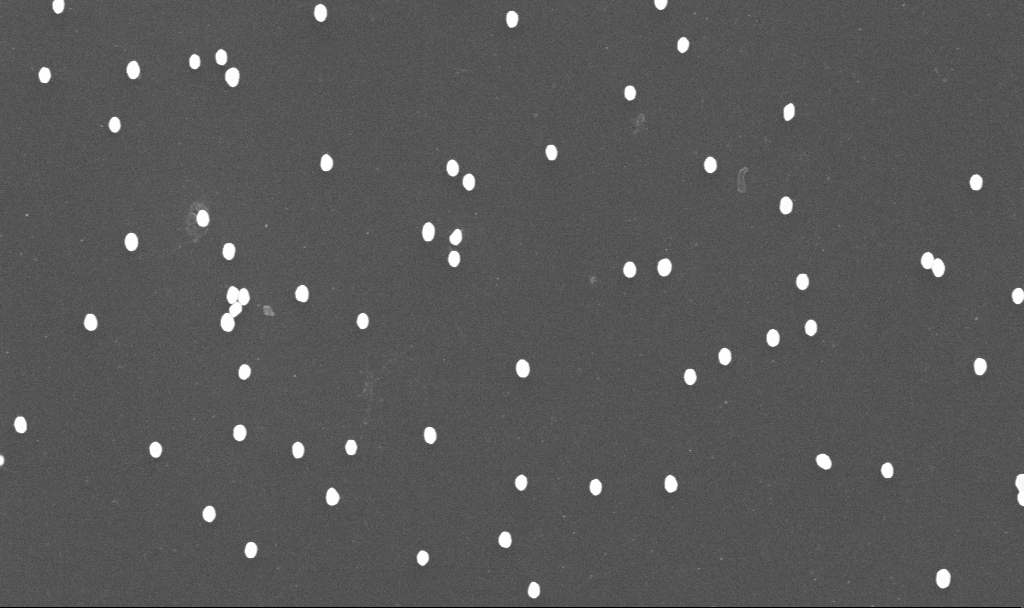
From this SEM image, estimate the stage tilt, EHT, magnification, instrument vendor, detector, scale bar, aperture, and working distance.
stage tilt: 0°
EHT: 10 kV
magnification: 70 K X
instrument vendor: Zeiss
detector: InLens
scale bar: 1000 nm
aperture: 30 µm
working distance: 3.4 mm